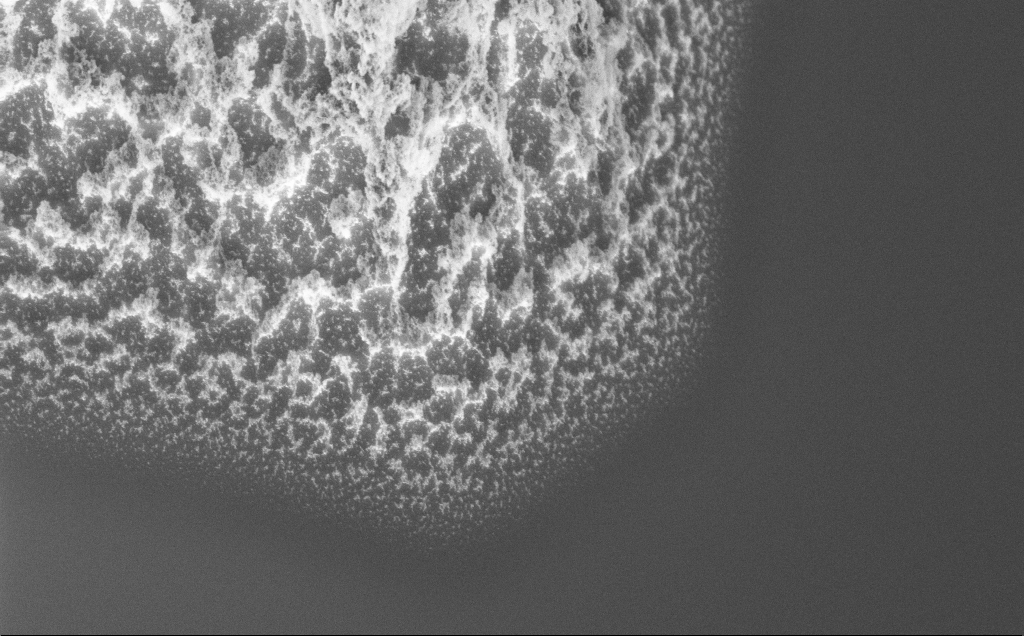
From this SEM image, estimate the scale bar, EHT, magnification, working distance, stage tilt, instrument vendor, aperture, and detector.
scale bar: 1000 nm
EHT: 5 kV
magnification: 36.66 K X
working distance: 8 mm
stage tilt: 45°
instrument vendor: Zeiss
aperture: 30 µm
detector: InLens